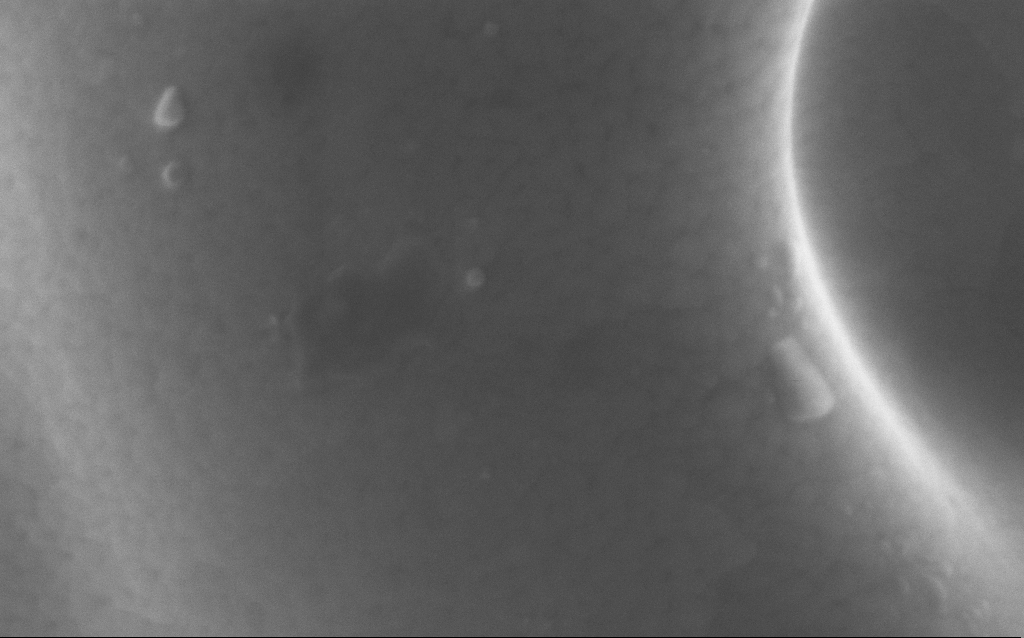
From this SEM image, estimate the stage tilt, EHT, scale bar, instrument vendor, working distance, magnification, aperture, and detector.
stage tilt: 0°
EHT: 5 kV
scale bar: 200 nm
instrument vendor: Zeiss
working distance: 3 mm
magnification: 331.72 K X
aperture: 30 µm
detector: InLens